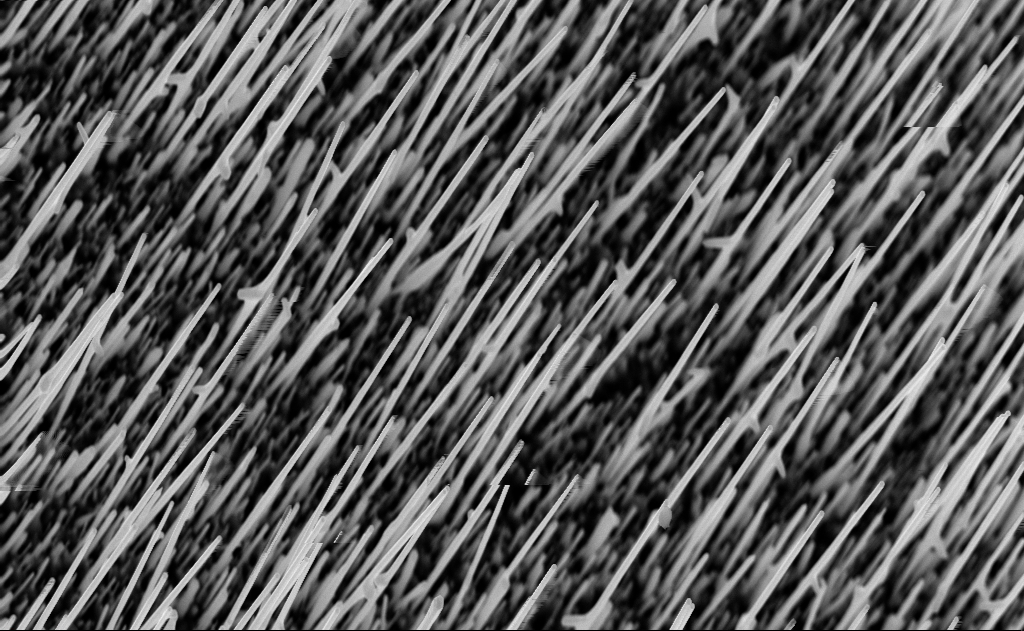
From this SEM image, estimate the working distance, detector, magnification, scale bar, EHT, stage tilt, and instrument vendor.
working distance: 5 mm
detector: InLens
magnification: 20 K X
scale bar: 2000 nm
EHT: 10 kV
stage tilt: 0°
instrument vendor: Zeiss